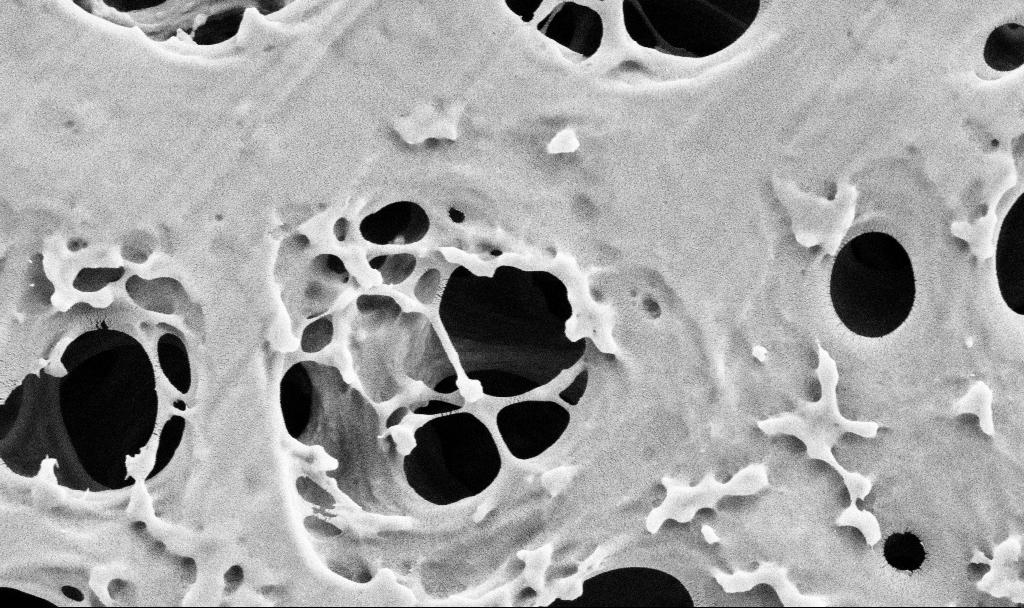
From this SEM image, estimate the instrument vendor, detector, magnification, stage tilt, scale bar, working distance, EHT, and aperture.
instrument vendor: Zeiss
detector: SE2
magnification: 50 K X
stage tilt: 0°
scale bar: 1000 nm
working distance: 3.7 mm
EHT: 2 kV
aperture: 30 µm